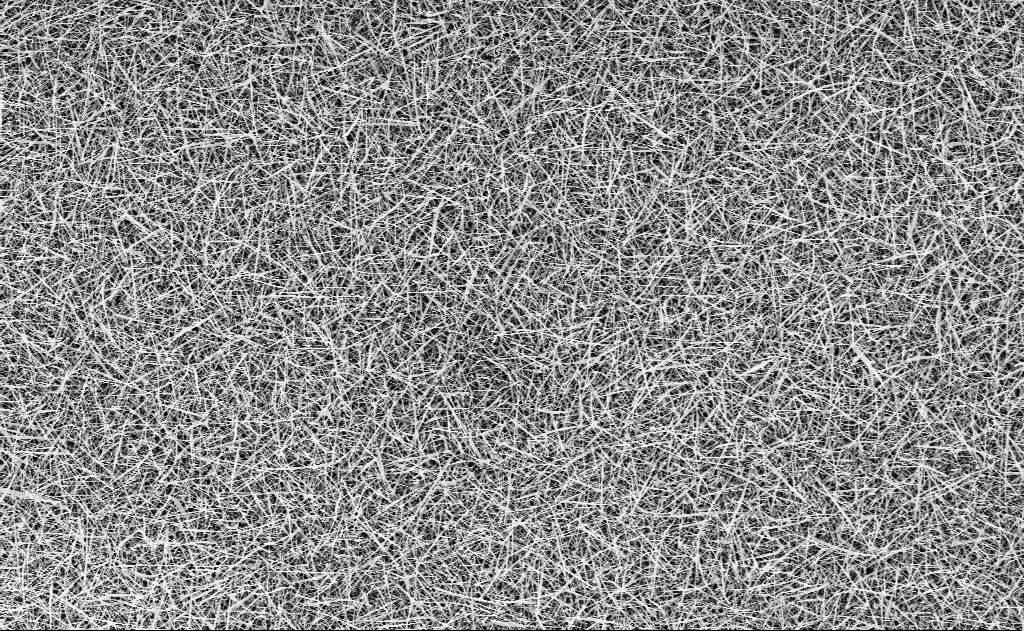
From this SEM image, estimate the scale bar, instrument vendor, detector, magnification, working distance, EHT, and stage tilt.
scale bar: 10000 nm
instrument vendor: Zeiss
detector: InLens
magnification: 5 K X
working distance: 15 mm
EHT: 10 kV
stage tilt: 0°